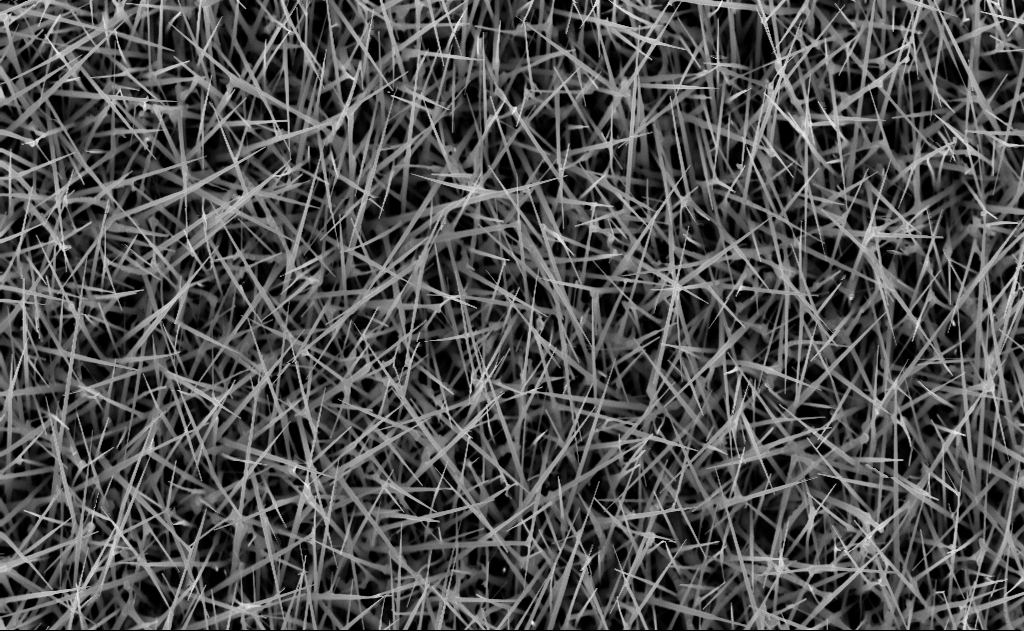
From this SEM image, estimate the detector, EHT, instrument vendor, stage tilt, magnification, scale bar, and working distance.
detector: InLens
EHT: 10 kV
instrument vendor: Zeiss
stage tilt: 0°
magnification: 20 K X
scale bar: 2000 nm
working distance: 7 mm